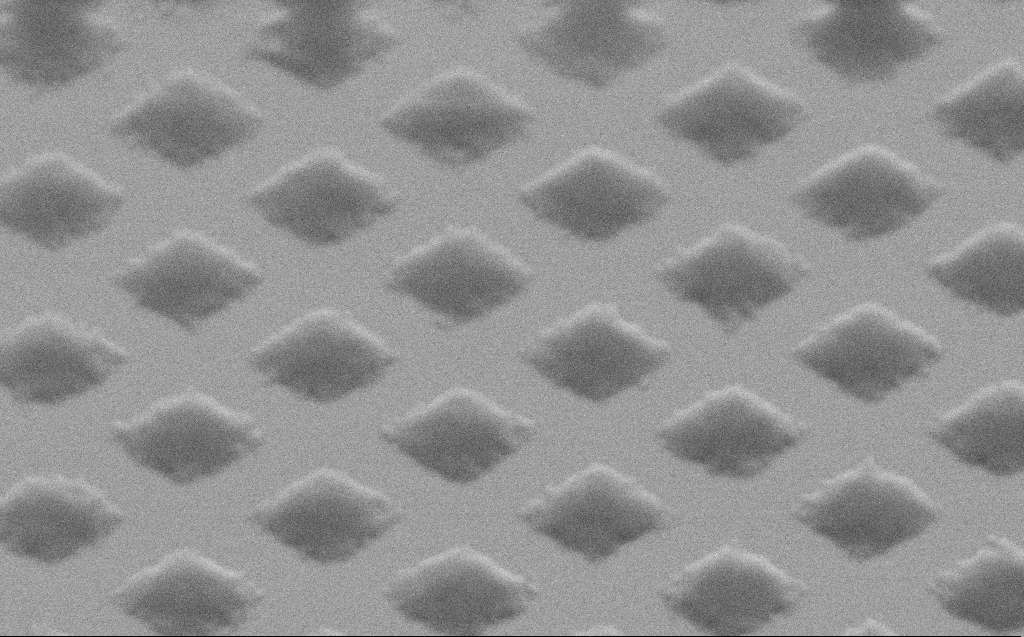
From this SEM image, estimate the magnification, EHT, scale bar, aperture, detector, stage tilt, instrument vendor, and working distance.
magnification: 20.13 K X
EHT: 3 kV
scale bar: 2000 nm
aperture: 30 µm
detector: SE2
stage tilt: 45°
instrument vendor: Zeiss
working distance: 5 mm